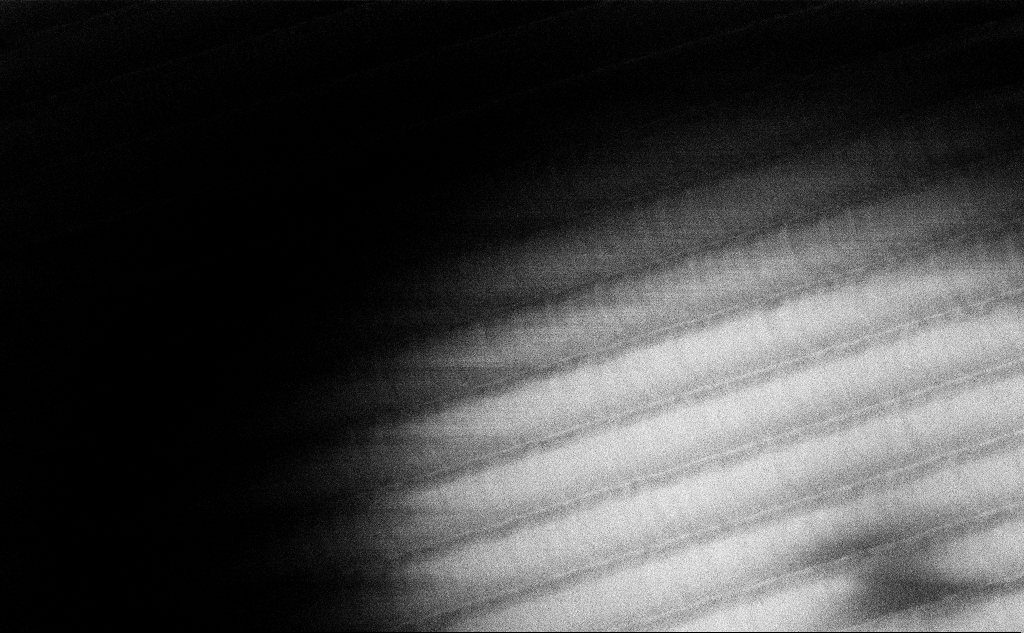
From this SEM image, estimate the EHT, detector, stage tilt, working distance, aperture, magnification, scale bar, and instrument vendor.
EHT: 3 kV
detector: InLens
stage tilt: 45°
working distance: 10.4 mm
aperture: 30 µm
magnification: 17.72 K X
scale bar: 2000 nm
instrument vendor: Zeiss